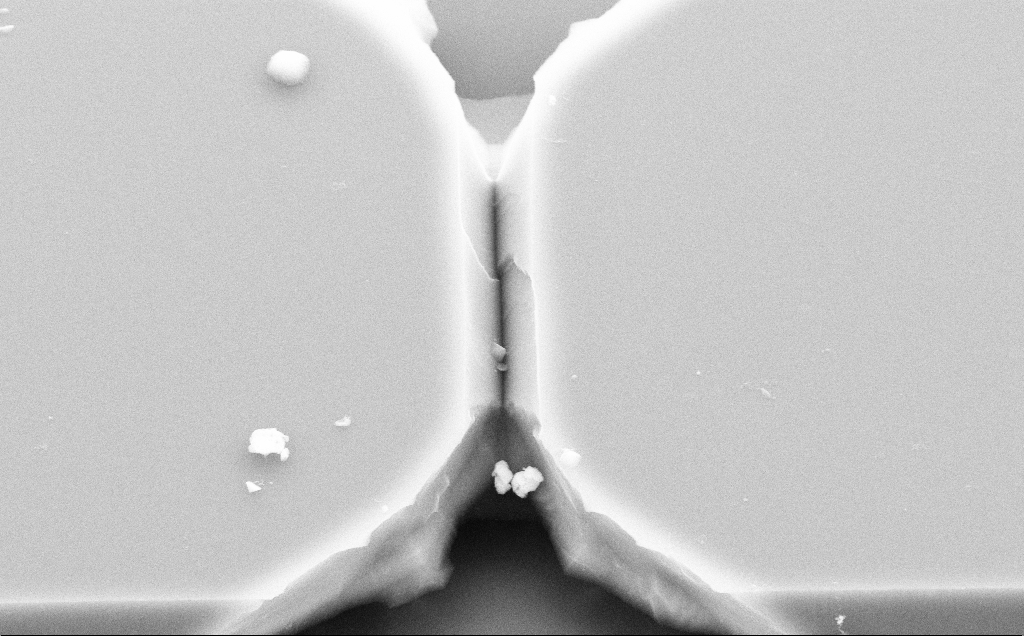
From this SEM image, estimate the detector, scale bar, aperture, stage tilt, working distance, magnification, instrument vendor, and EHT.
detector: SE2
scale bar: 1000 nm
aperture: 30 µm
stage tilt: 31.9°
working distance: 10 mm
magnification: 14.68 K X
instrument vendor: Zeiss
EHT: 10 kV